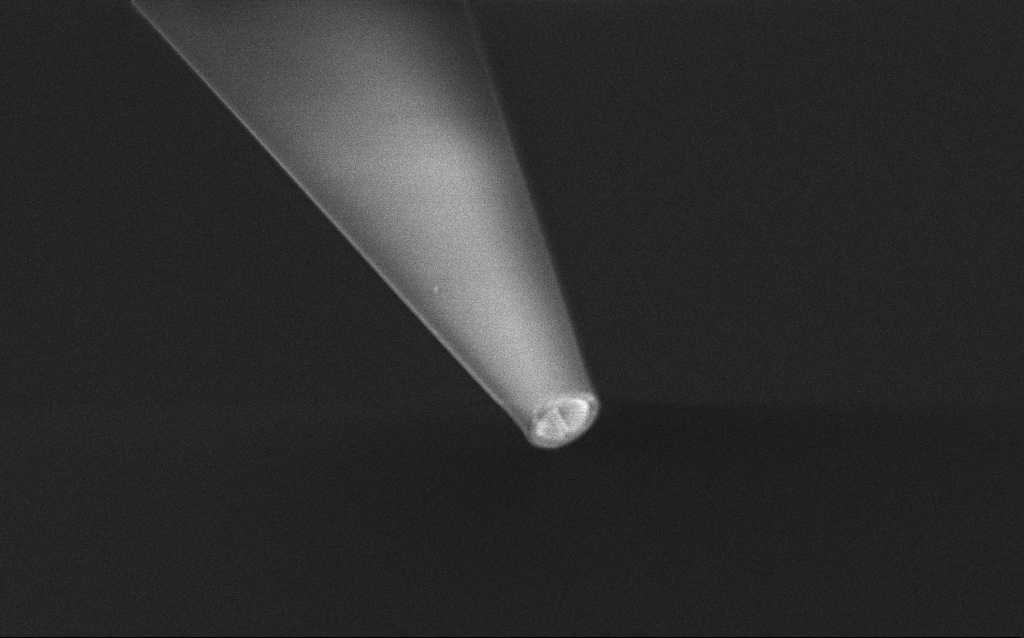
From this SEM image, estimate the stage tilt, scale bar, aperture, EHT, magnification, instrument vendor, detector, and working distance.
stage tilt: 45°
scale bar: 2000 nm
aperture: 30 µm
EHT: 1 kV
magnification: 10 K X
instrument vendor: Zeiss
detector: InLens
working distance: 7 mm